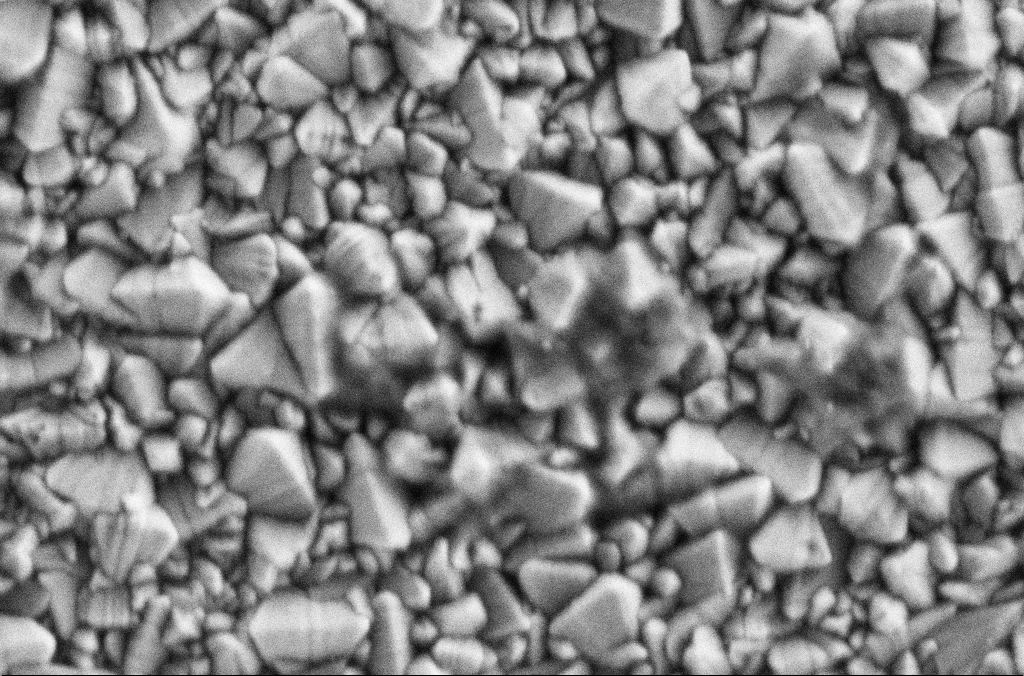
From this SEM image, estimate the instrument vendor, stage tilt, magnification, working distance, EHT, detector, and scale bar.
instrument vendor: Zeiss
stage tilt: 0°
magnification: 100 K X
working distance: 1.9 mm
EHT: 2 kV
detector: SE2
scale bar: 200 nm